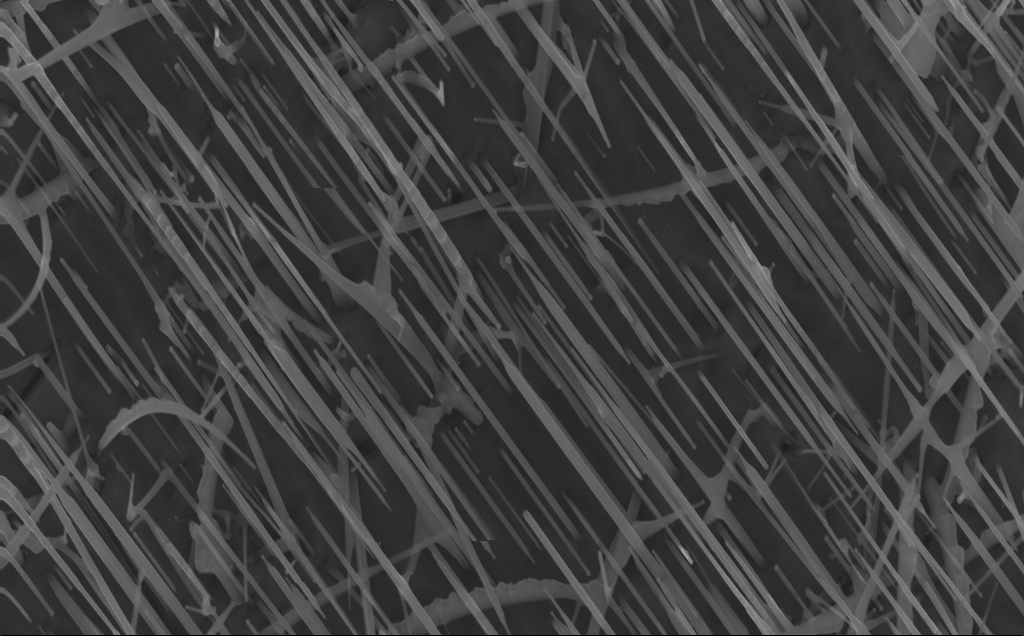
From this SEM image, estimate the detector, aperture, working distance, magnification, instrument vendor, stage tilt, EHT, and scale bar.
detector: InLens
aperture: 30 µm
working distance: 4 mm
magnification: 40 K X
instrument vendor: Zeiss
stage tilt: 0°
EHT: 10 kV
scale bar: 1000 nm